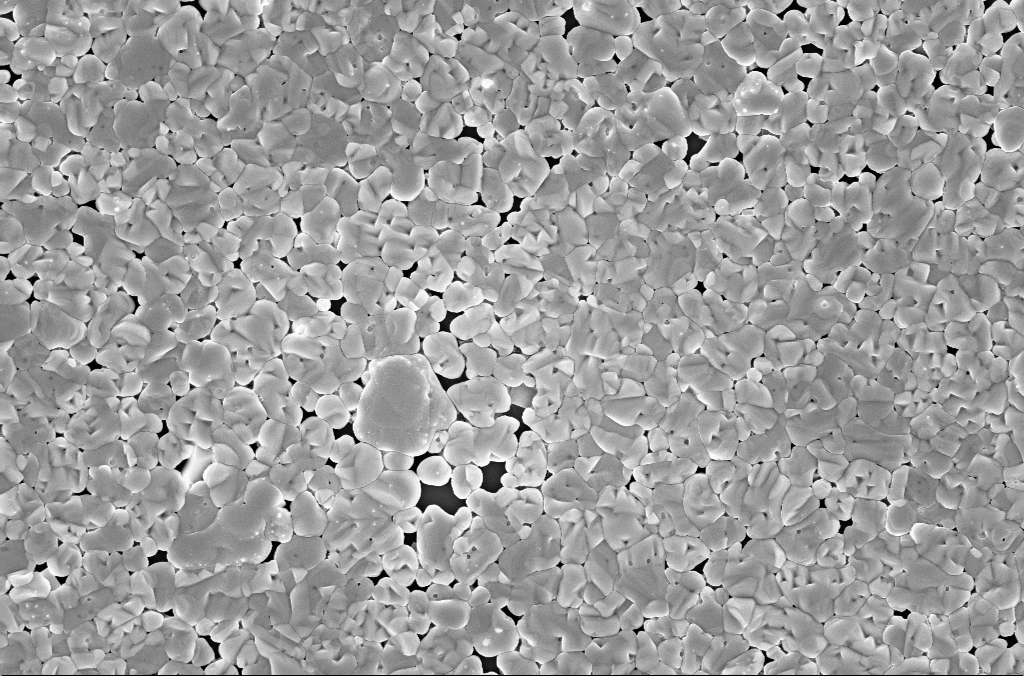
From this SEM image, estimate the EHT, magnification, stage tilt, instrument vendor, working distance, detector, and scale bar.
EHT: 5 kV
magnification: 20 K X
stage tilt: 0°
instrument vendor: Zeiss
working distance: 3 mm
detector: InLens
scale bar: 2000 nm